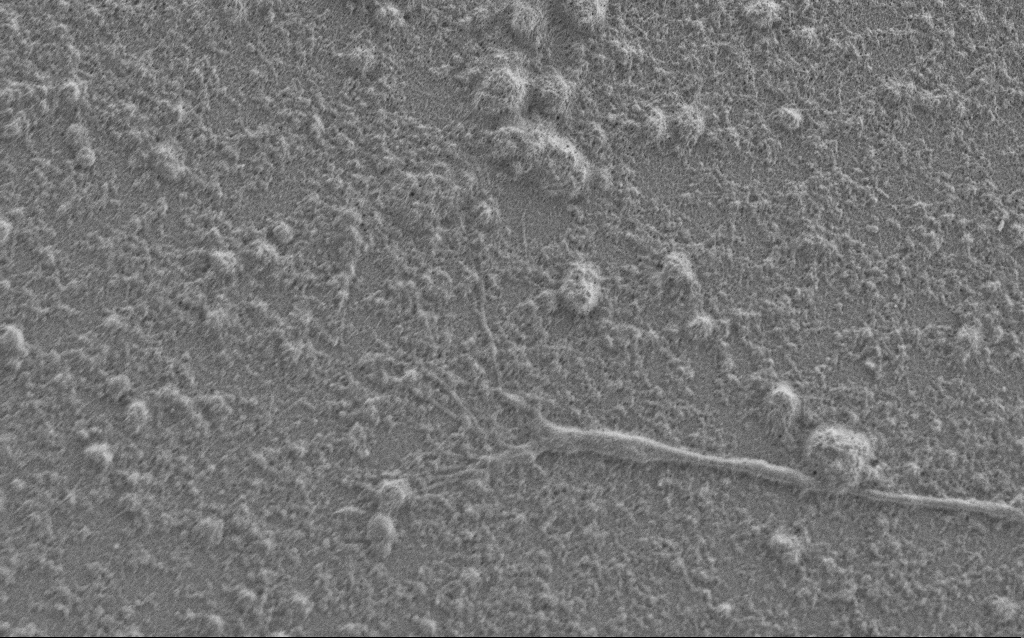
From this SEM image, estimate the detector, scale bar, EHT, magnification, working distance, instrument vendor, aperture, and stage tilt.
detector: SE2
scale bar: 2000 nm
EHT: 1 kV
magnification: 7.5 K X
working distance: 6 mm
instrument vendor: Zeiss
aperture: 30 µm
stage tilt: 0°